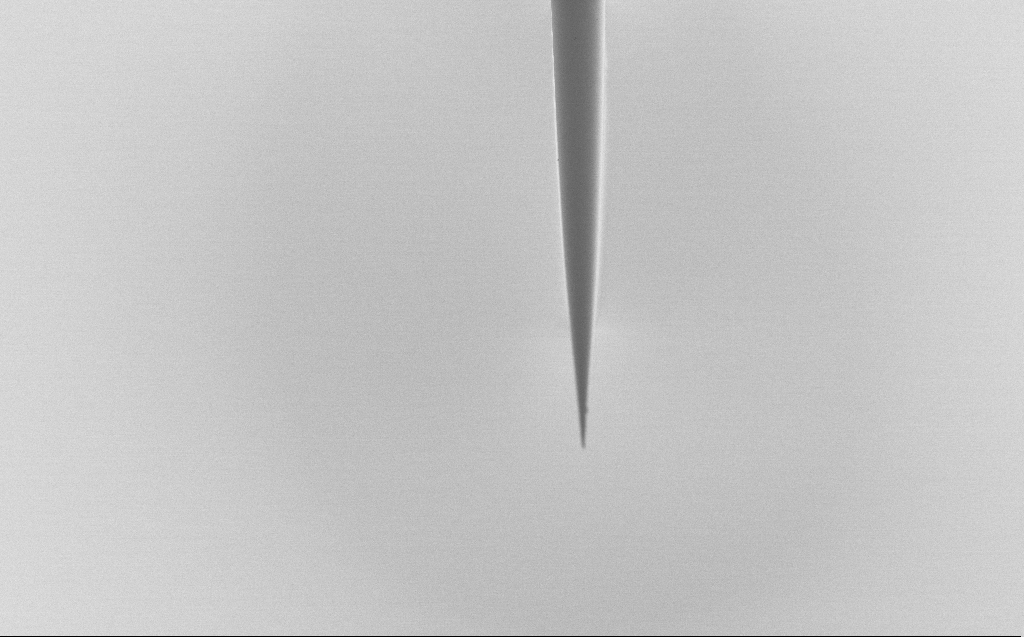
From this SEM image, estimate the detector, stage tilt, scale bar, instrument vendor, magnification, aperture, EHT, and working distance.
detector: SE2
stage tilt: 45°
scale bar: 20000 nm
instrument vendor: Zeiss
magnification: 1 K X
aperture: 30 µm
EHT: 1 kV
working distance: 5 mm